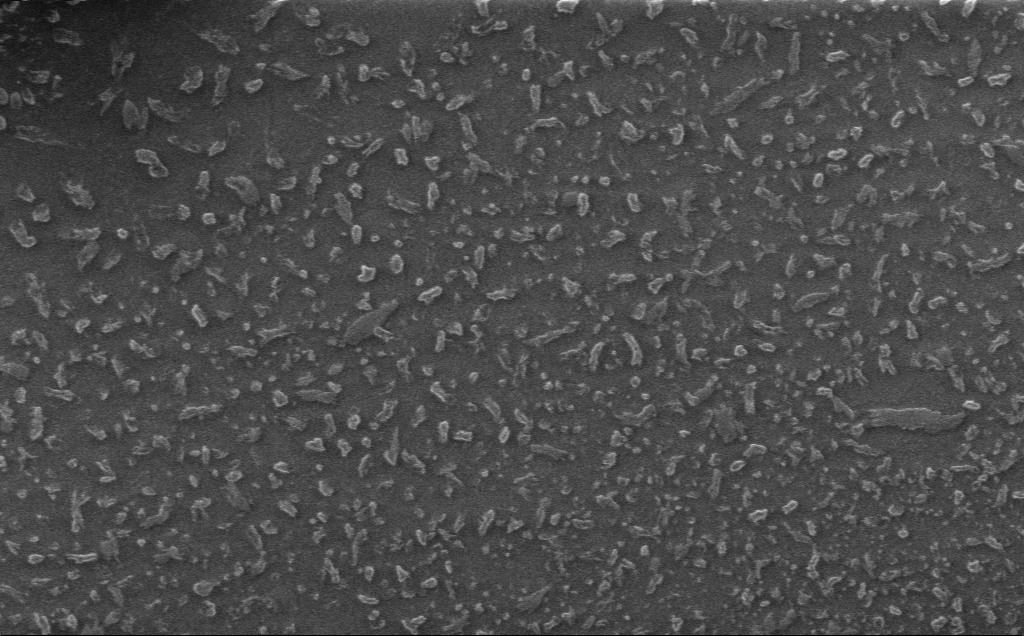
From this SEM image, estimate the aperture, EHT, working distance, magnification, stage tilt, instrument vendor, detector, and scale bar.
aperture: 30 µm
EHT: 1 kV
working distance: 3 mm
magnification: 5.1 K X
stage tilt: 0°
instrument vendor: Zeiss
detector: InLens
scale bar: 10000 nm